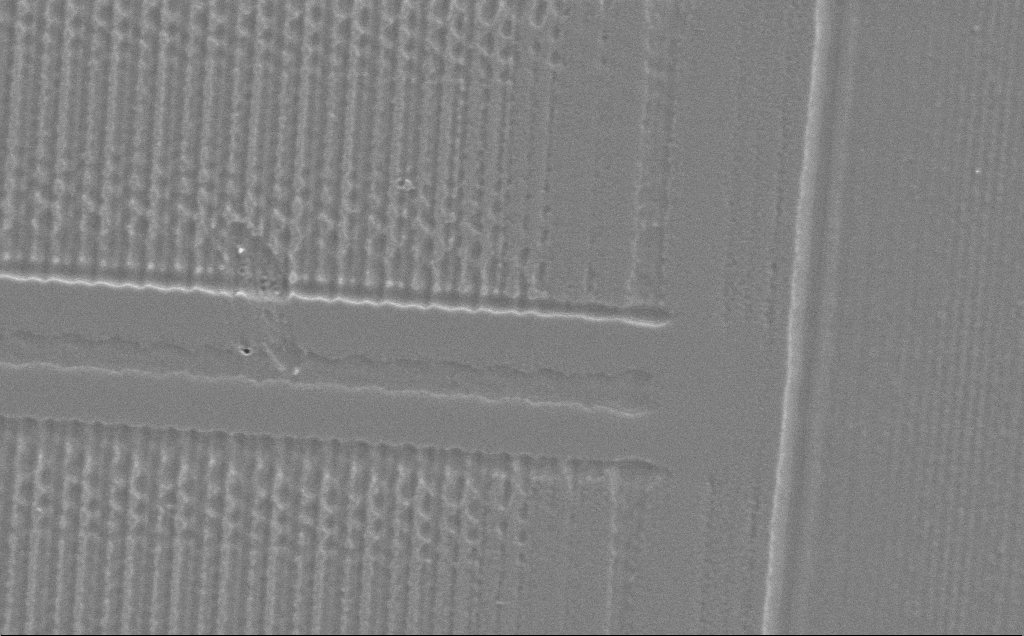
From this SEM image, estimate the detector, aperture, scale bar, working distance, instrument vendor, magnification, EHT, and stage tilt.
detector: SE2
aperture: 30 µm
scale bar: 10000 nm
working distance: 10 mm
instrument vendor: Zeiss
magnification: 4.37 K X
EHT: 5 kV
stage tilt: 0°